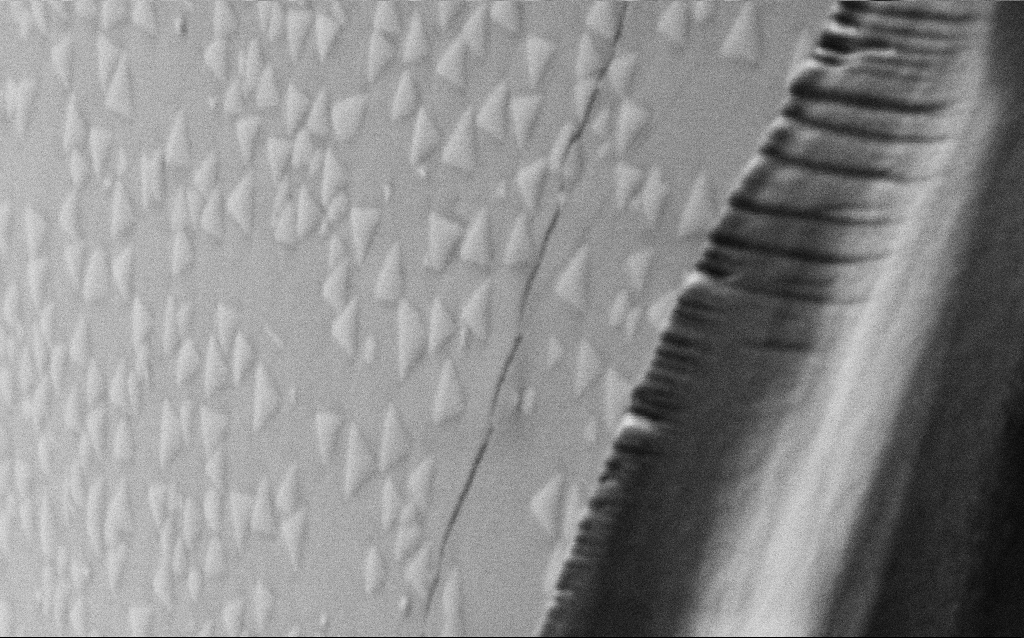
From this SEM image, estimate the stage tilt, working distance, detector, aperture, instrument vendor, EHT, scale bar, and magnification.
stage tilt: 45°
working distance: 6.6 mm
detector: SE2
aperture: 30 µm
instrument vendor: Zeiss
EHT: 1 kV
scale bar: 200 nm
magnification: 150 K X